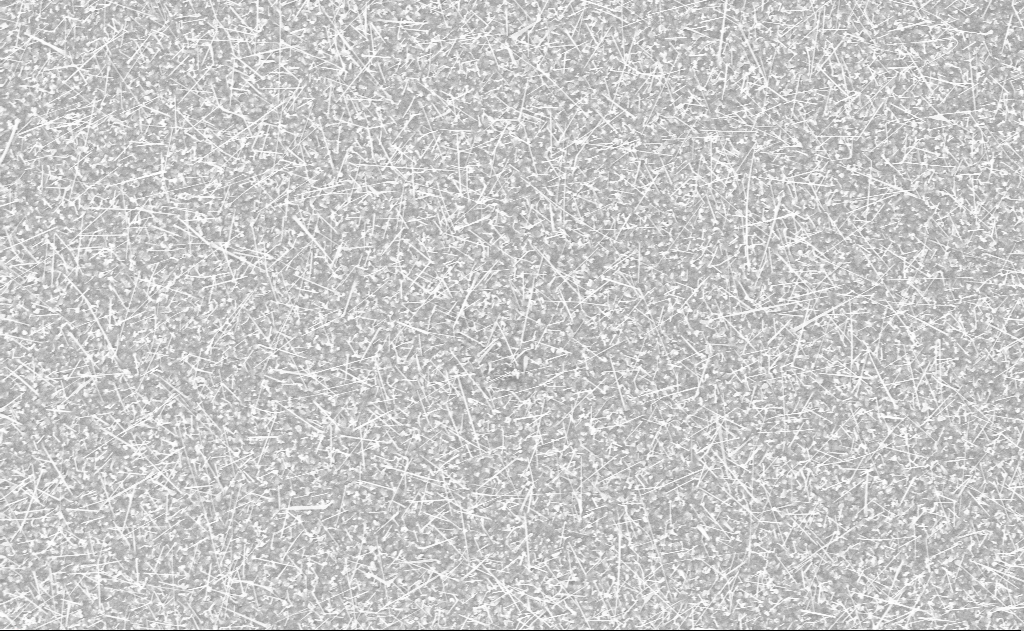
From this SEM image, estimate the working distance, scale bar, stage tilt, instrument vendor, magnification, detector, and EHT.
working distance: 20 mm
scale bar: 2000 nm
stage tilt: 0°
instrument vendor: Zeiss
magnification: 10 K X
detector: InLens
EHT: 10 kV